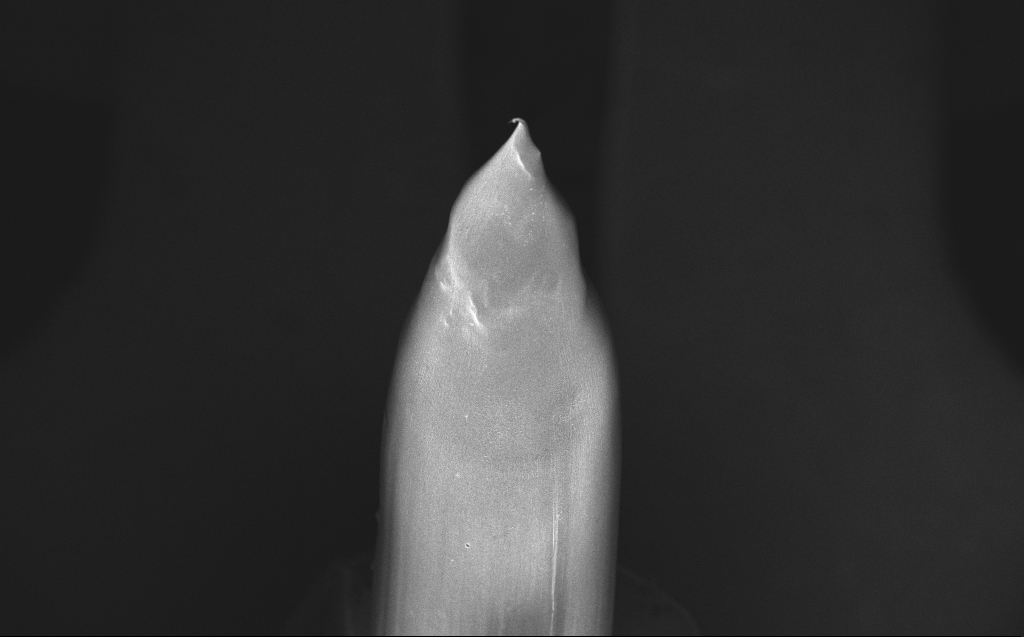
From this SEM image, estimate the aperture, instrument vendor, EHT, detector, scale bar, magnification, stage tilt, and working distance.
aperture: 30 µm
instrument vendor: Zeiss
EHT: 10 kV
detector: InLens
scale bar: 200000 nm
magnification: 0.185 K X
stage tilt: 40°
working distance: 4 mm